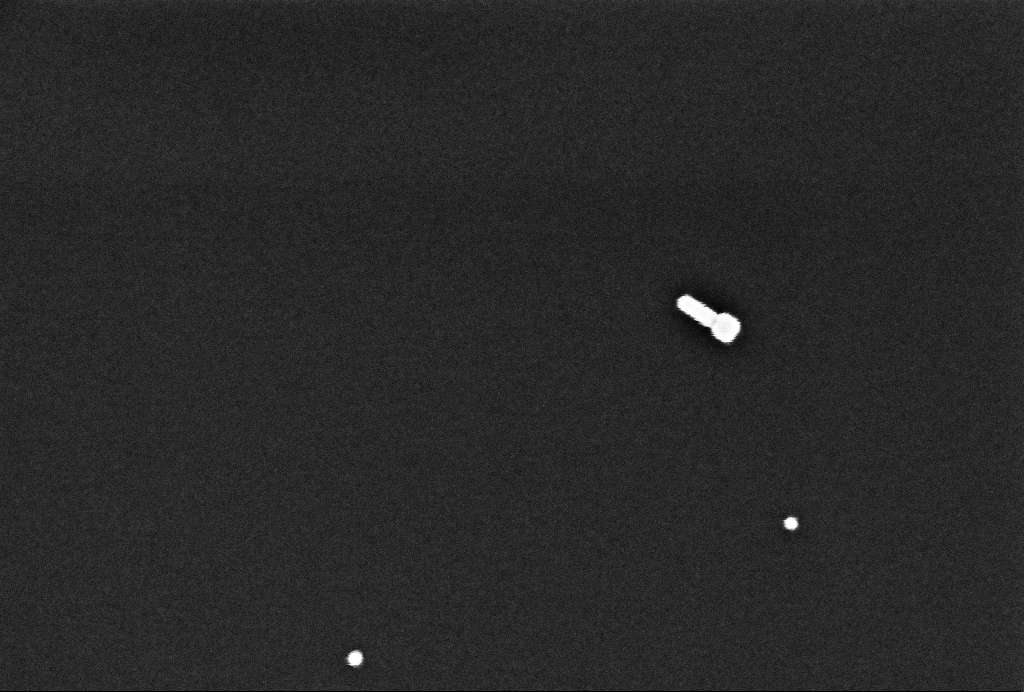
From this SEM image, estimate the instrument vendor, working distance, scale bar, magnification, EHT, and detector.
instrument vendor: Zeiss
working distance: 3.3 mm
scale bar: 200 nm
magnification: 214.8 K X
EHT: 2 kV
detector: InLens